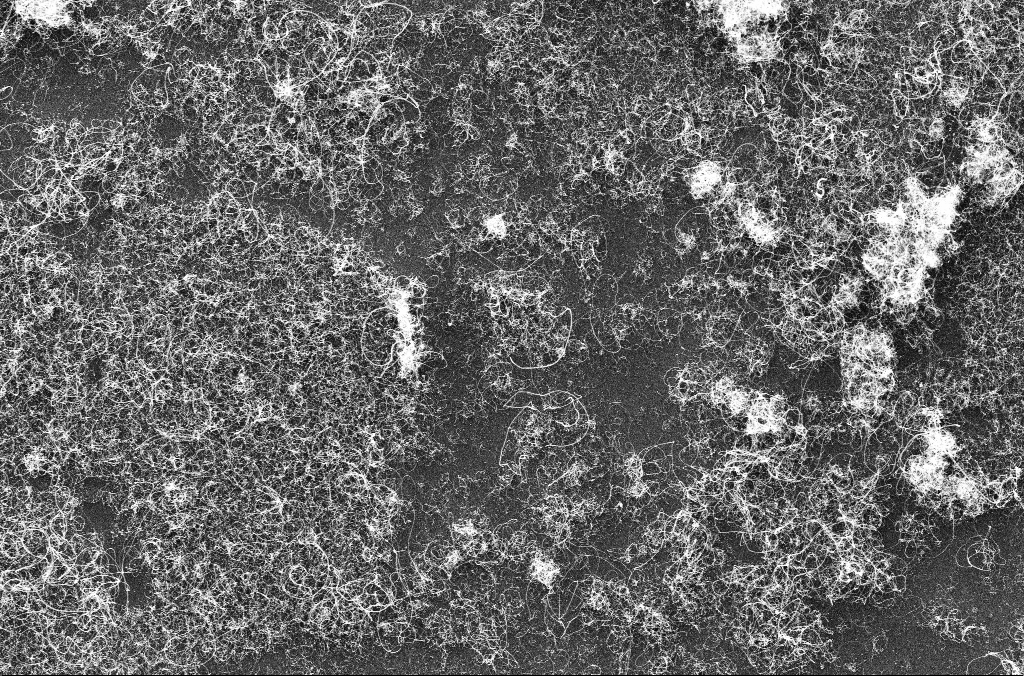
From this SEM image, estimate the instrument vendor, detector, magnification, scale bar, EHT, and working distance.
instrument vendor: Zeiss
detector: InLens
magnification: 5 K X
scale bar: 10000 nm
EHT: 10 kV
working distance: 3.3 mm